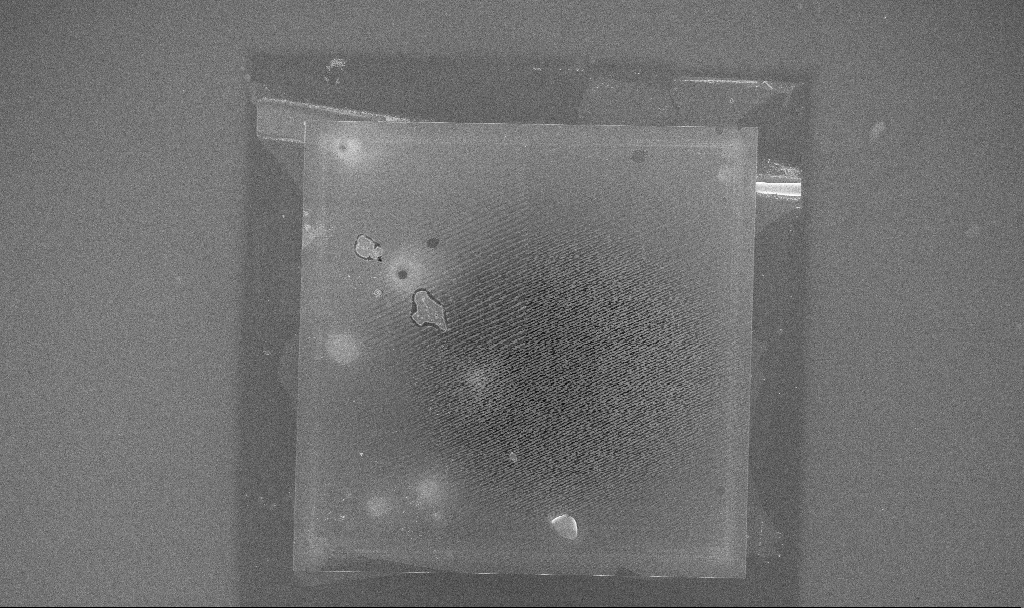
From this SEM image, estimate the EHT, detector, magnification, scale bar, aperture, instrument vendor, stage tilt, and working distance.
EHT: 5 kV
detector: InLens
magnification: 0.168 K X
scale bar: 200000 nm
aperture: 30 µm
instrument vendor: Zeiss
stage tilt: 0°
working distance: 4.2 mm